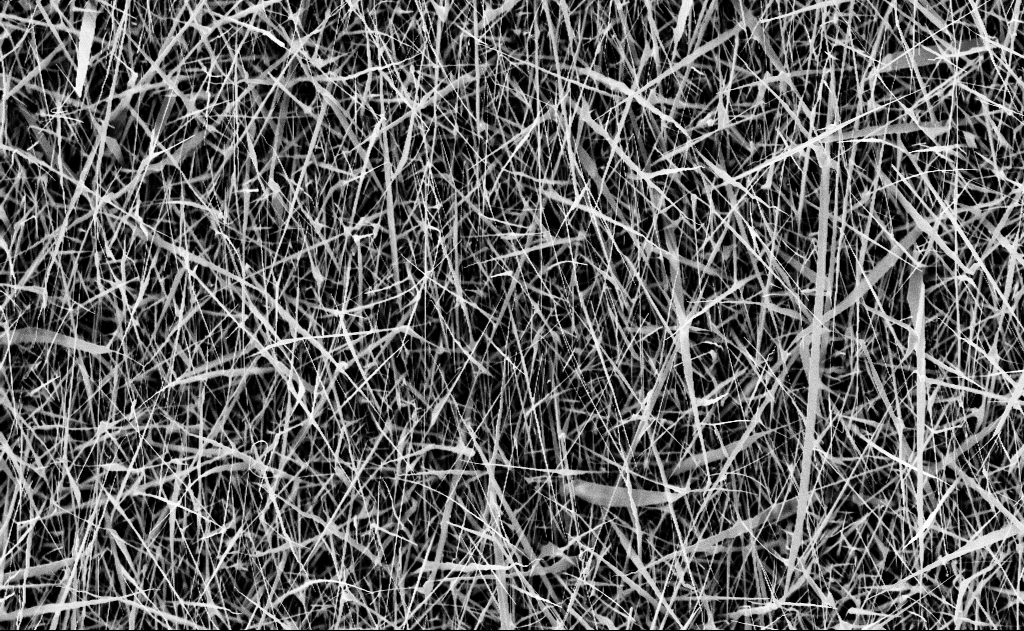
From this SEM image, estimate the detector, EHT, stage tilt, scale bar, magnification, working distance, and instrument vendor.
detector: InLens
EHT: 10 kV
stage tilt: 0°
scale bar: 2000 nm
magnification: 10 K X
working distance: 17 mm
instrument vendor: Zeiss